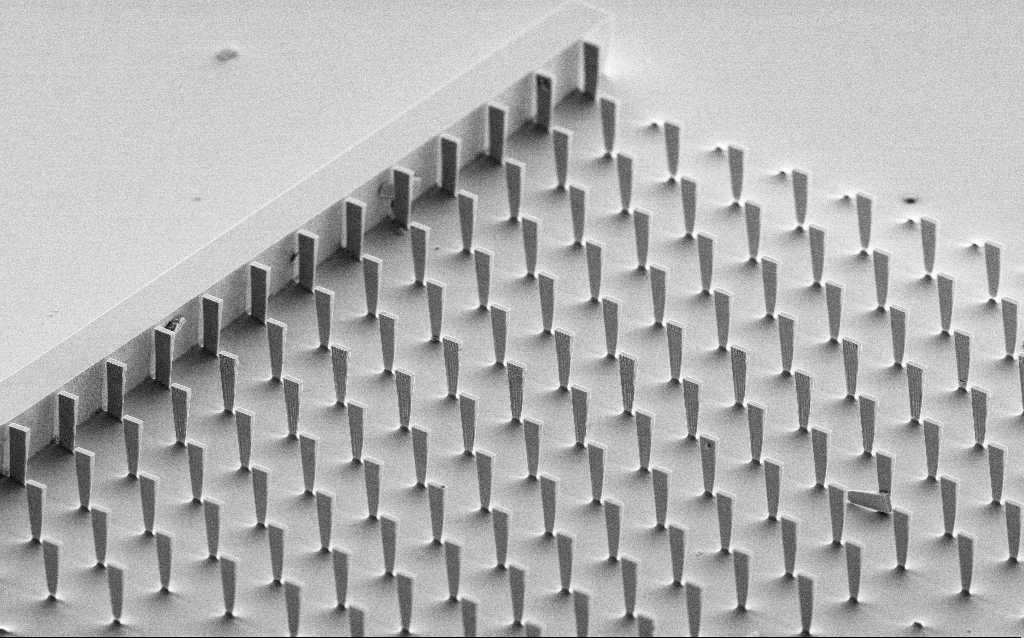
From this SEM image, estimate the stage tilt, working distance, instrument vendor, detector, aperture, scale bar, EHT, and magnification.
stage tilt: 60°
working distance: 9.7 mm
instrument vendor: Zeiss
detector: SE2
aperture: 30 µm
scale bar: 20000 nm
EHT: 5 kV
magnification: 0.981 K X